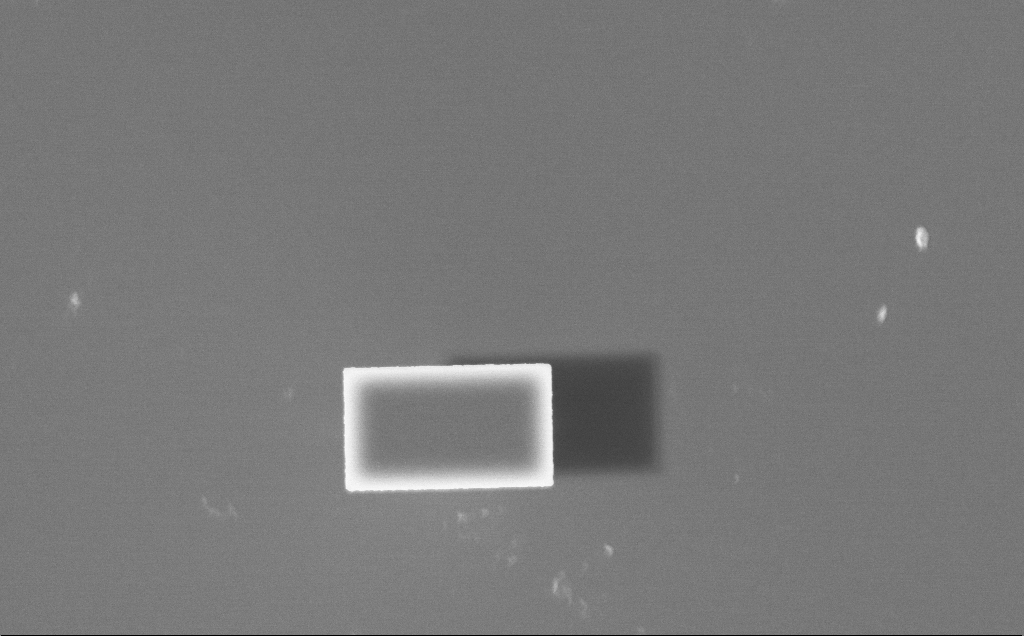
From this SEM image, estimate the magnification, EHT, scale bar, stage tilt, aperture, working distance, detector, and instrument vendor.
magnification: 15.79 K X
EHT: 7.5 kV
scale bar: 2000 nm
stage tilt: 0°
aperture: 30 µm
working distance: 4 mm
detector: InLens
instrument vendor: Zeiss